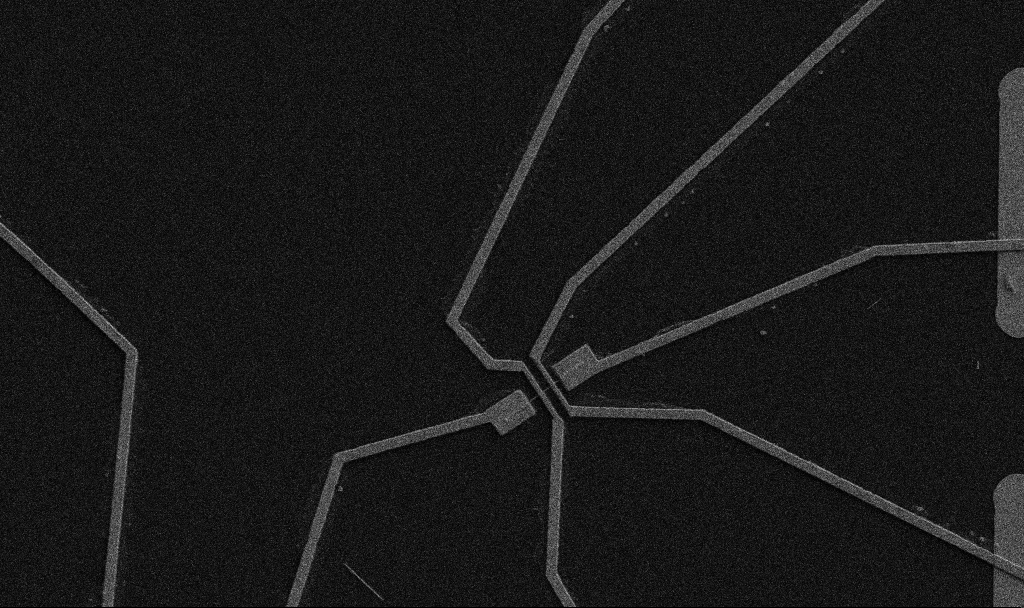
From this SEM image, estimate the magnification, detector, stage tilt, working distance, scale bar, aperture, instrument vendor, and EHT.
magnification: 5 K X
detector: SE2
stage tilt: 0°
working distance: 10.7 mm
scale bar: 10000 nm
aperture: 30 µm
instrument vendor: Zeiss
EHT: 5 kV